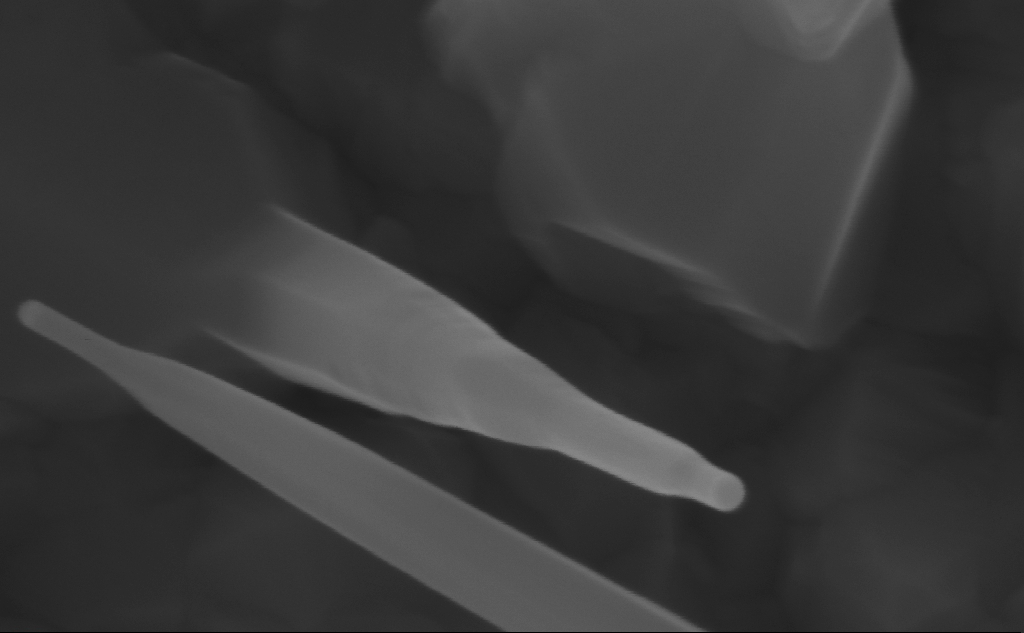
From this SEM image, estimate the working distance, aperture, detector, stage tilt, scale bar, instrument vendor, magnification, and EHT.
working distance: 7 mm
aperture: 30 µm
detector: InLens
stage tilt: -0°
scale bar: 200 nm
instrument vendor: Zeiss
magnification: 351.18 K X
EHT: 10 kV